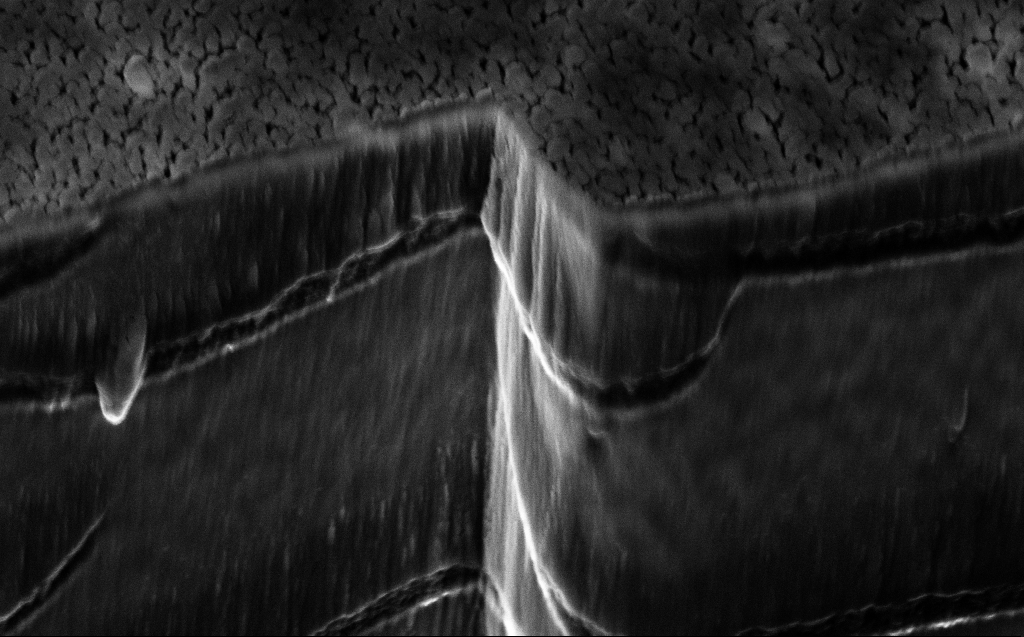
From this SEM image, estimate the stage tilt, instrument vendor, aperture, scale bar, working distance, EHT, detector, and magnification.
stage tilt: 45°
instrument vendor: Zeiss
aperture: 30 µm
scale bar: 1000 nm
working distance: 4 mm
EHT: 5 kV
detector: InLens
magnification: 39.98 K X